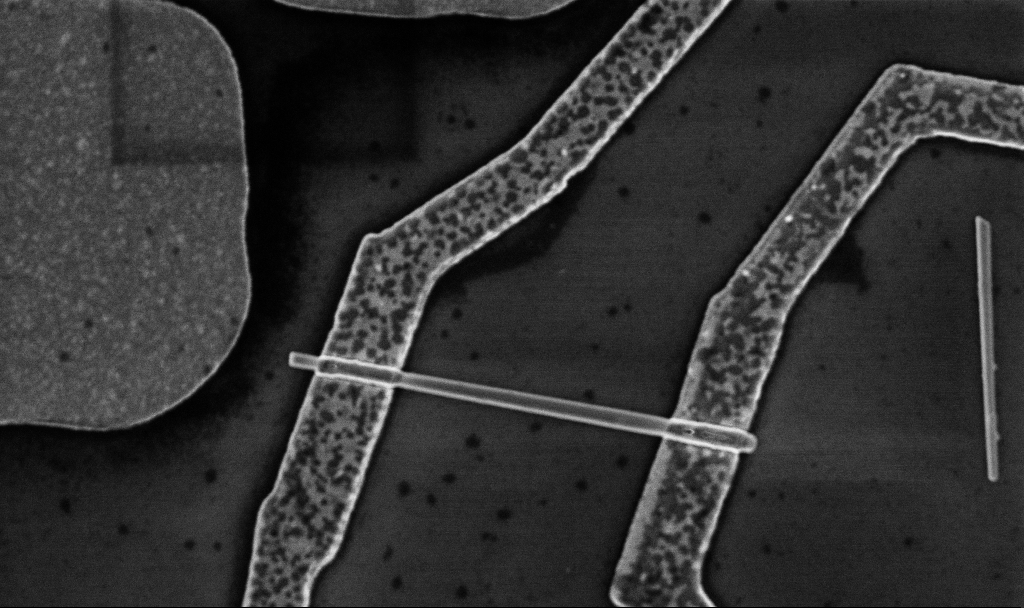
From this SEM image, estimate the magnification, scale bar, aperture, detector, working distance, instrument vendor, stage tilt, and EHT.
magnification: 30 K X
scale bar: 1000 nm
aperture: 30 µm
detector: InLens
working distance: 8.7 mm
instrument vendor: Zeiss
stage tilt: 0°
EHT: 5 kV